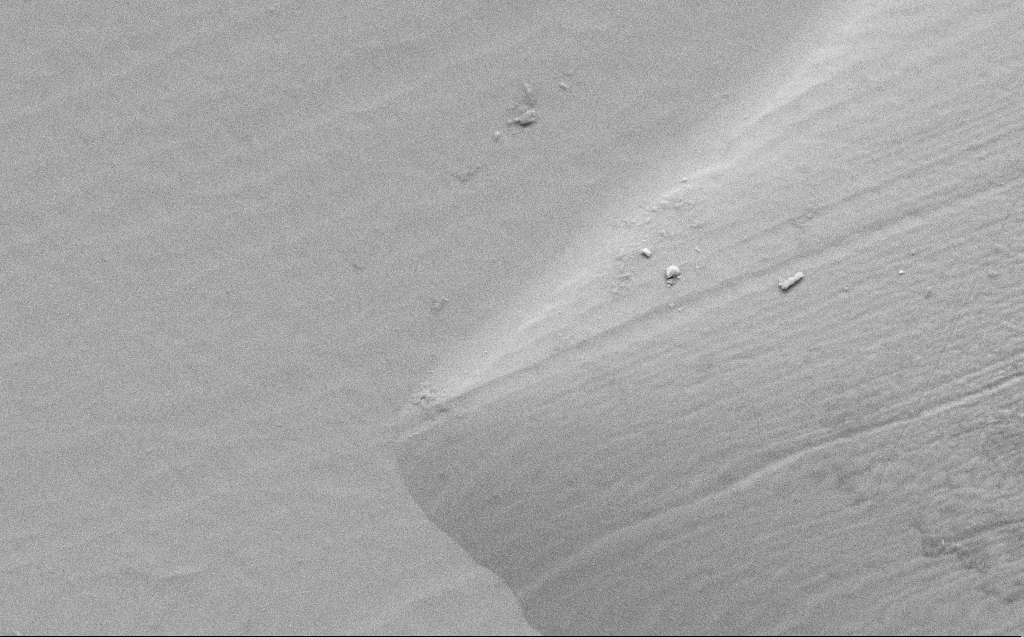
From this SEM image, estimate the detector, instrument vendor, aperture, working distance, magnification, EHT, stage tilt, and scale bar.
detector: SE2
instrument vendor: Zeiss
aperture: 30 µm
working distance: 8 mm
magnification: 5.85 K X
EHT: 5 kV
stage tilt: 45°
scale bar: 10000 nm